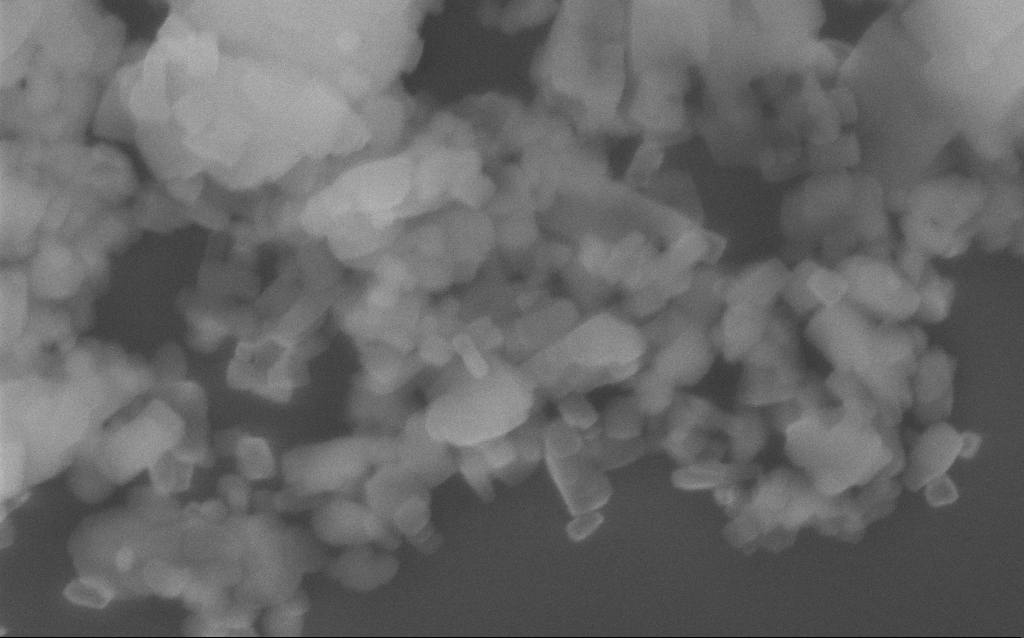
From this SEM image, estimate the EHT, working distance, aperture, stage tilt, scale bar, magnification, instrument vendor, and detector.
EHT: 10 kV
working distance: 3 mm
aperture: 30 µm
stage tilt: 0°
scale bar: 200 nm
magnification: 151.53 K X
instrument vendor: Zeiss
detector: InLens